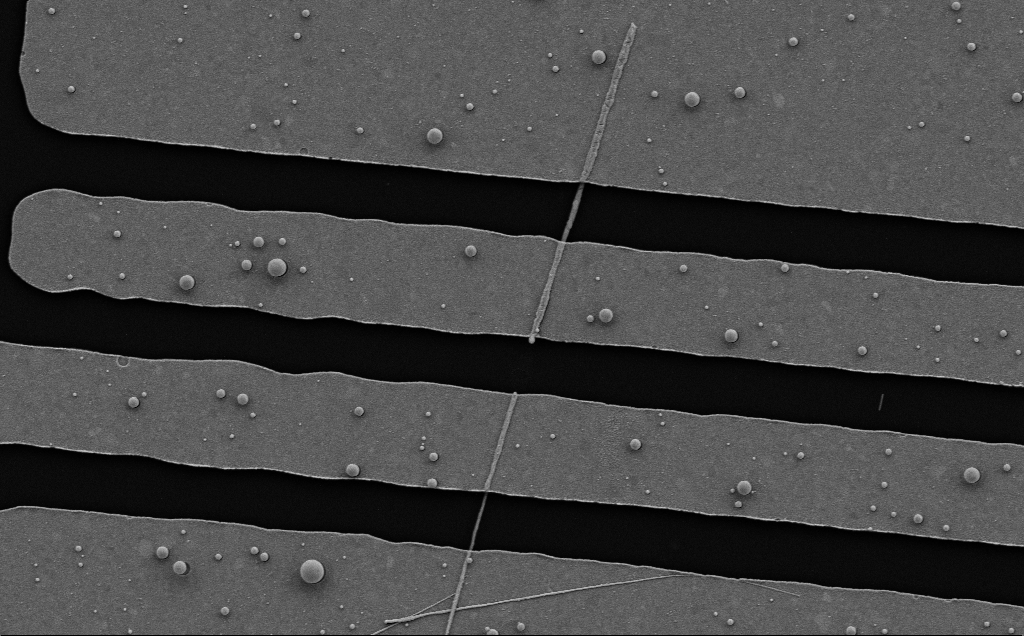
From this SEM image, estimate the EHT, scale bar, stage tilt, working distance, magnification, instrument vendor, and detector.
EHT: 5 kV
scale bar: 2000 nm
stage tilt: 0°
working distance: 8 mm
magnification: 14.46 K X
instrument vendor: Zeiss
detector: SE2